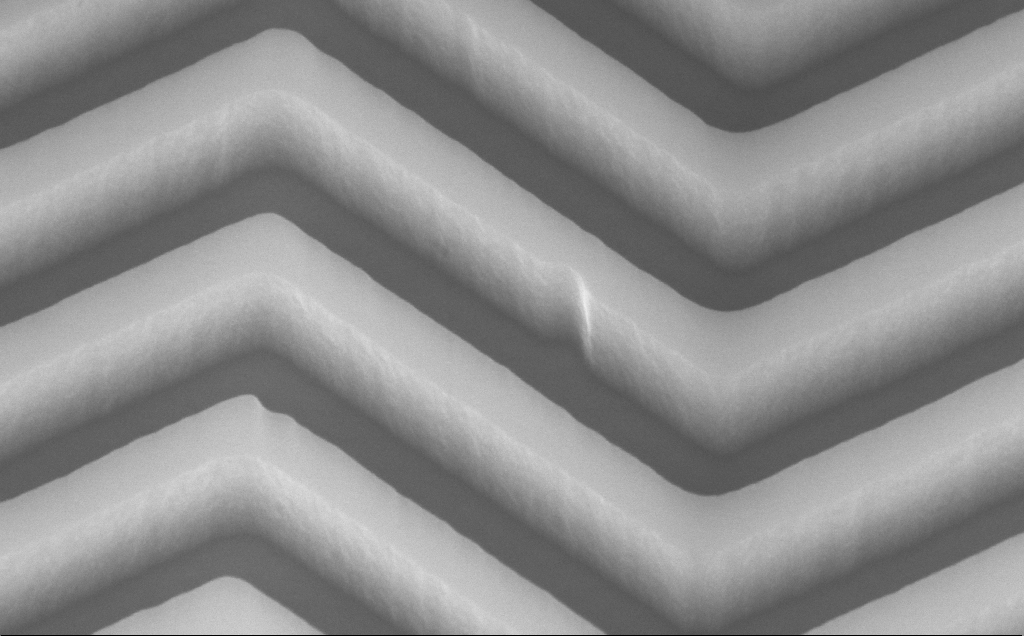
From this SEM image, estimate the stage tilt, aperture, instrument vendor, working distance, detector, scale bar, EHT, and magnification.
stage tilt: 45°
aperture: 30 µm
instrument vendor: Zeiss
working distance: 6 mm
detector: InLens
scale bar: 200 nm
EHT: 10 kV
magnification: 169.73 K X